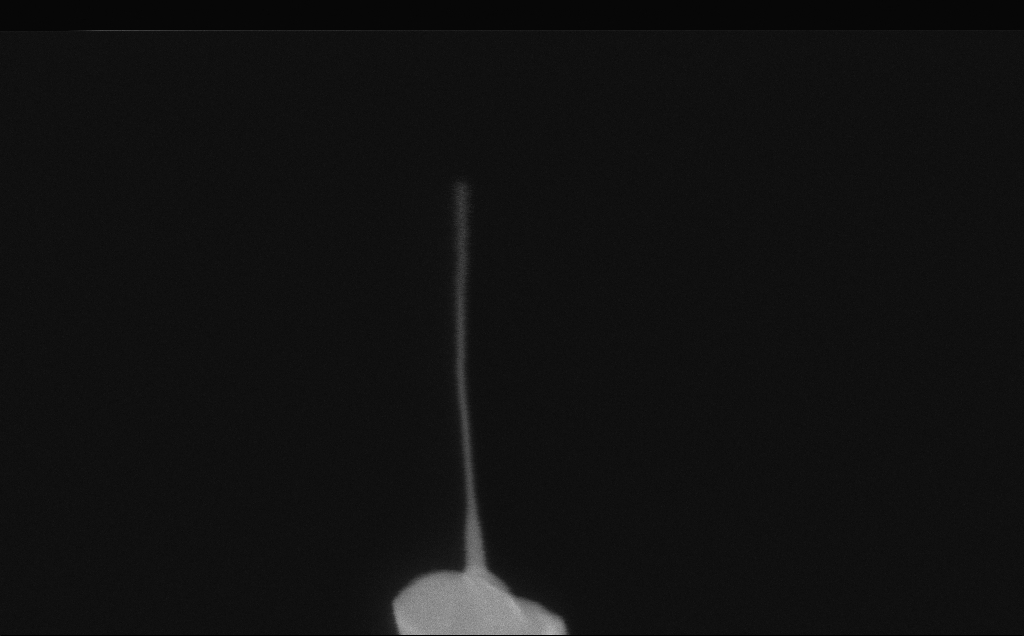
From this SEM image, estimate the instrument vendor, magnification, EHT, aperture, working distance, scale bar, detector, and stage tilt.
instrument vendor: Zeiss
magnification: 257.27 K X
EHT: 10 kV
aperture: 30 µm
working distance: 6 mm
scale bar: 200 nm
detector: InLens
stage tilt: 0°